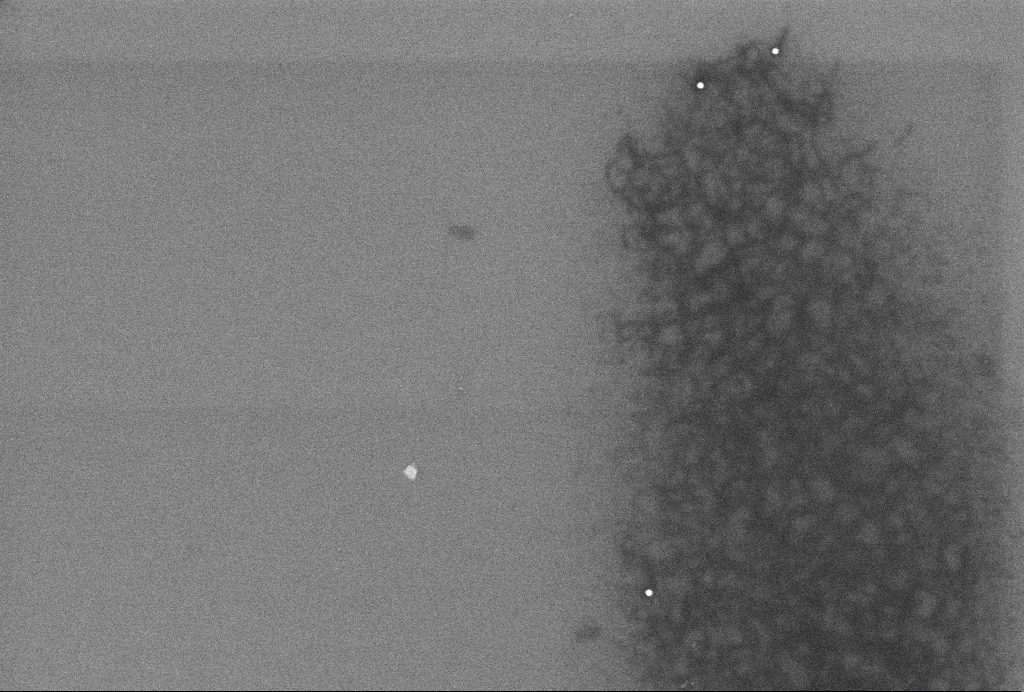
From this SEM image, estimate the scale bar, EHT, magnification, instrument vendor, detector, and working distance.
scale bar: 200 nm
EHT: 2 kV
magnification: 83.64 K X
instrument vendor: Zeiss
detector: InLens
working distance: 3.3 mm